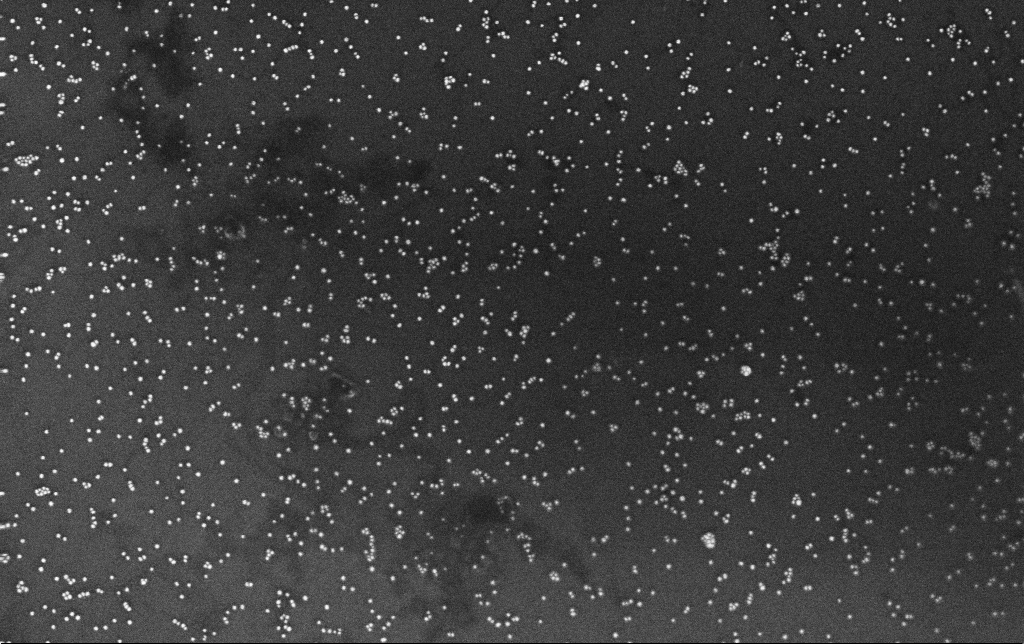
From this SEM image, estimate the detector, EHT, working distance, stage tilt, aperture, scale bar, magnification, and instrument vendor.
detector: InLens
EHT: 10 kV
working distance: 3.4 mm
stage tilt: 0°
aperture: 30 µm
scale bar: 200 nm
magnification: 100 K X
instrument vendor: Zeiss